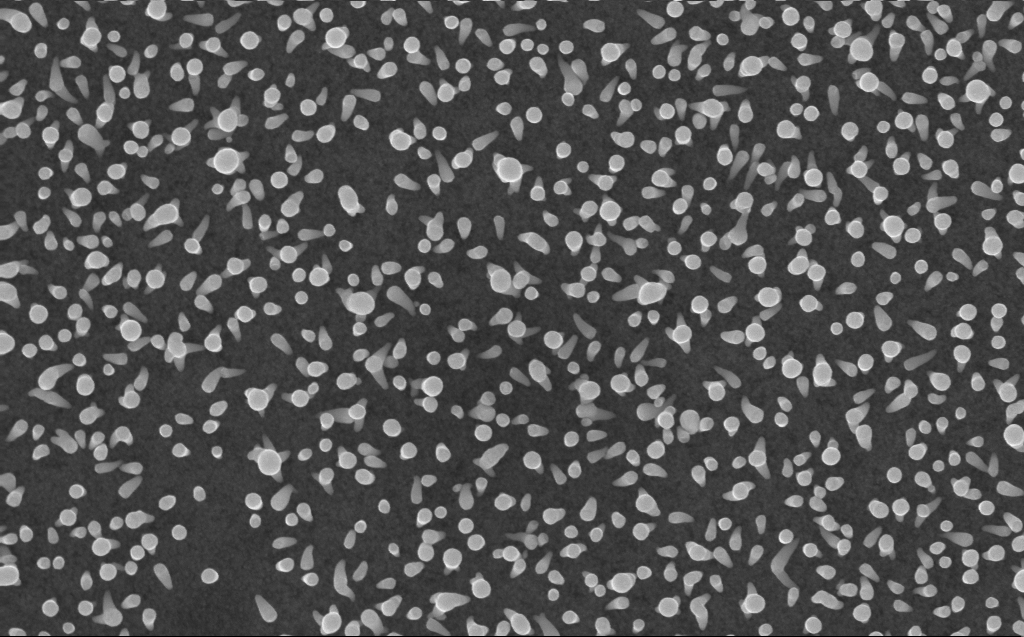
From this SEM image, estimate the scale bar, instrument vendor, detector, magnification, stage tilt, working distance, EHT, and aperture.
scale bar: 1000 nm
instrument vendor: Zeiss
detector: InLens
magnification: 50 K X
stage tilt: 0°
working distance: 3 mm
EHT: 10 kV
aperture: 30 µm